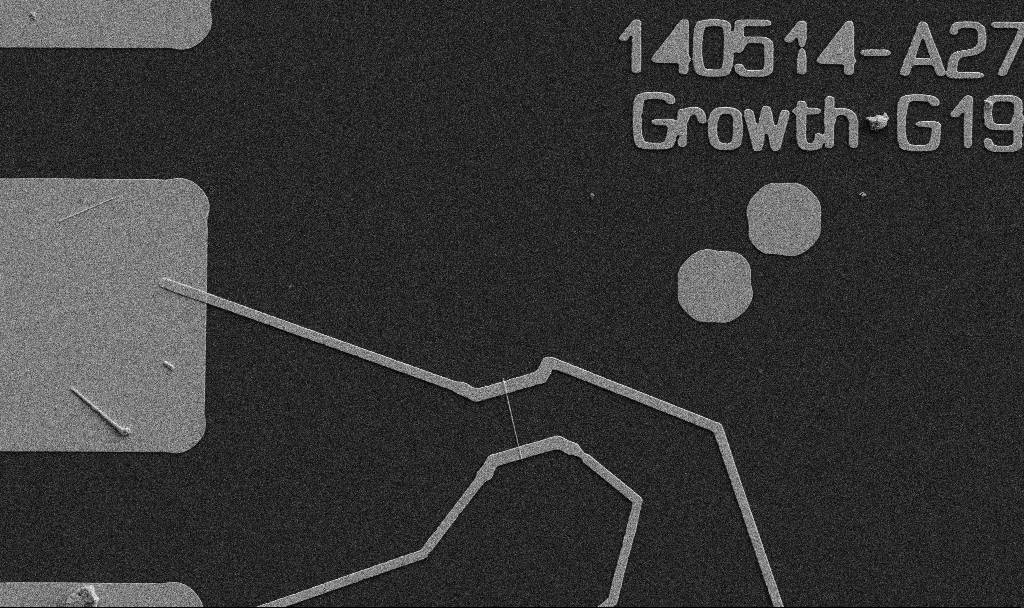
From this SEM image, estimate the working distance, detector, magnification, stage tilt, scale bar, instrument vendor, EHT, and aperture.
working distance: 10.7 mm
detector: SE2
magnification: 5 K X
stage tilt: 0°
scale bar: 10000 nm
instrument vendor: Zeiss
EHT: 5 kV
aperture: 30 µm